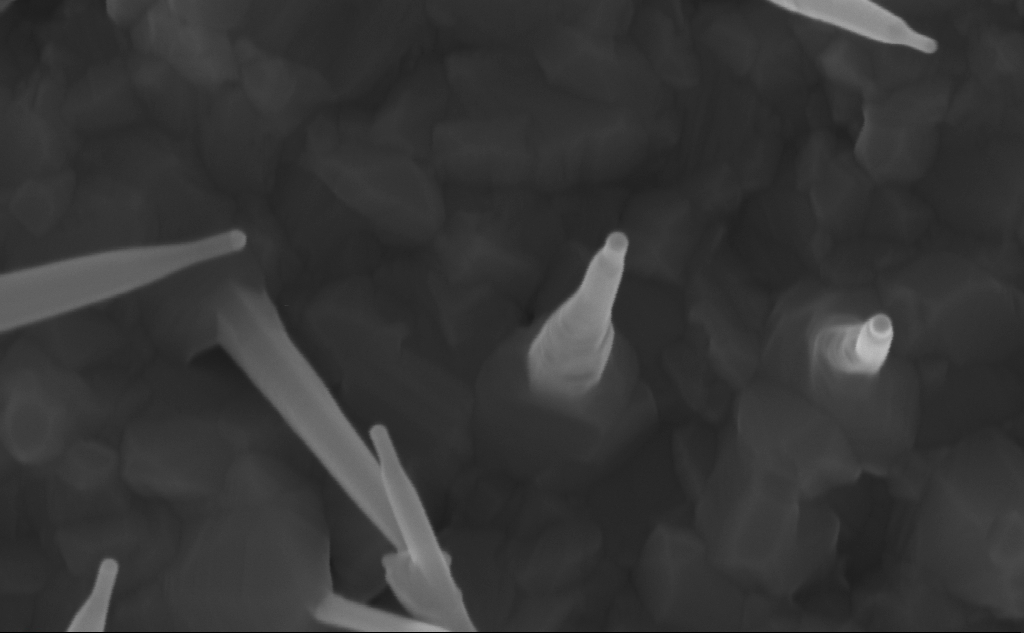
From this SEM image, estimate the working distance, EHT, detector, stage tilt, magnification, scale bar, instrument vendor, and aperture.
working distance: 7 mm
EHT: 10 kV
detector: InLens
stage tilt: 0°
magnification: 189.48 K X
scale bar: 100 nm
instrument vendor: Zeiss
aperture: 30 µm